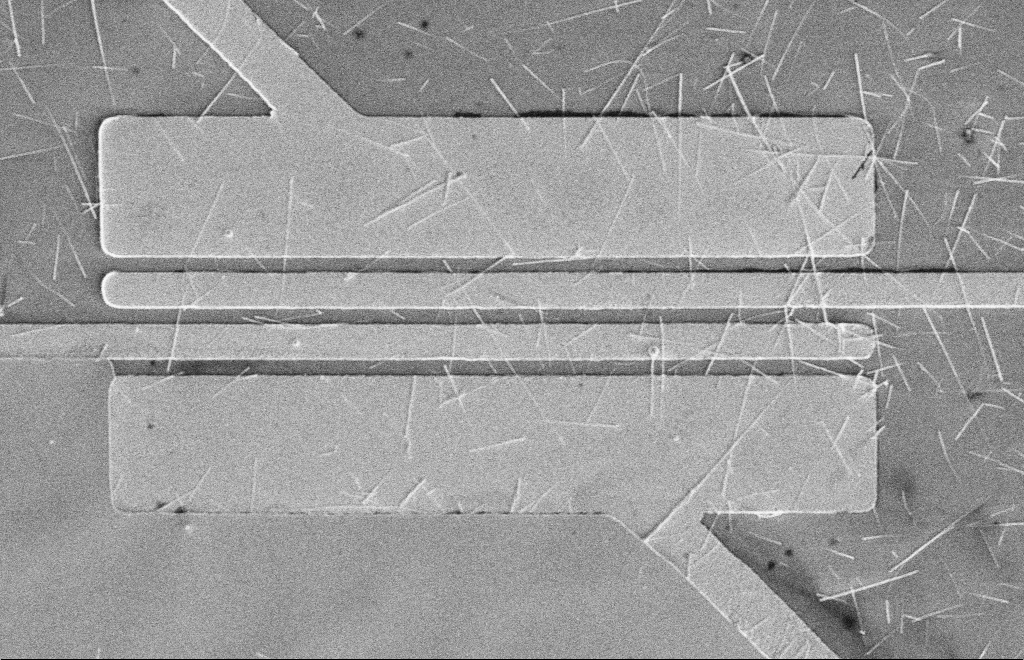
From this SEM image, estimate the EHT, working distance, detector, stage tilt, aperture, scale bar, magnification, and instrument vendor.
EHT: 5 kV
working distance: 16 mm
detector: SE2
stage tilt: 0°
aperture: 10 µm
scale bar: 10000 nm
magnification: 4.69 K X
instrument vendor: Zeiss